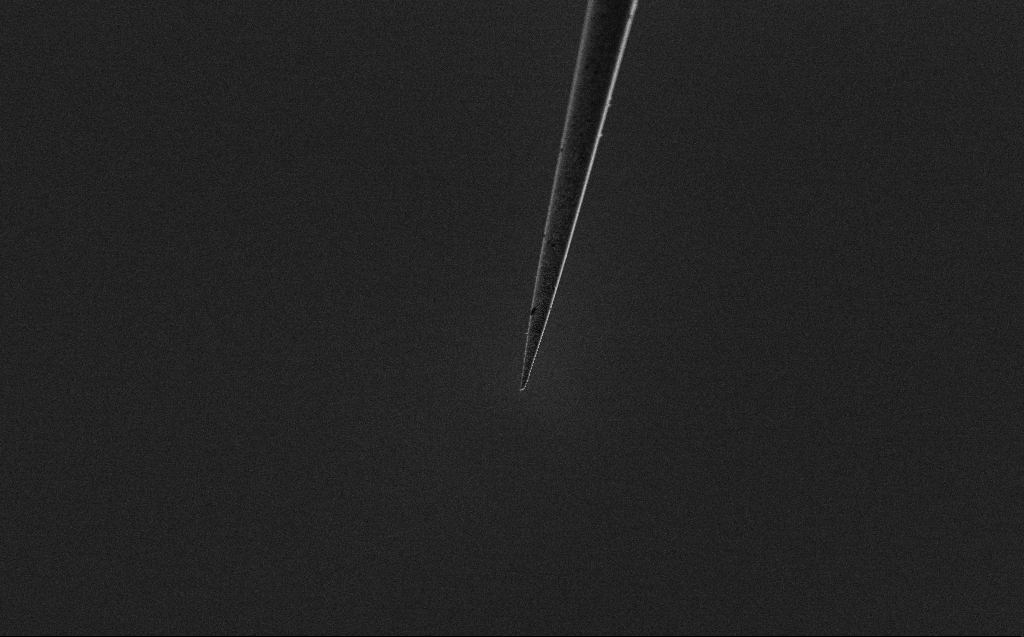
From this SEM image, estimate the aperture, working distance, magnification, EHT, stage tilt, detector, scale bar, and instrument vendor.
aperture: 30 µm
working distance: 4 mm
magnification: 5 K X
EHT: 2 kV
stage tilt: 45°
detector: SE2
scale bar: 10000 nm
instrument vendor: Zeiss